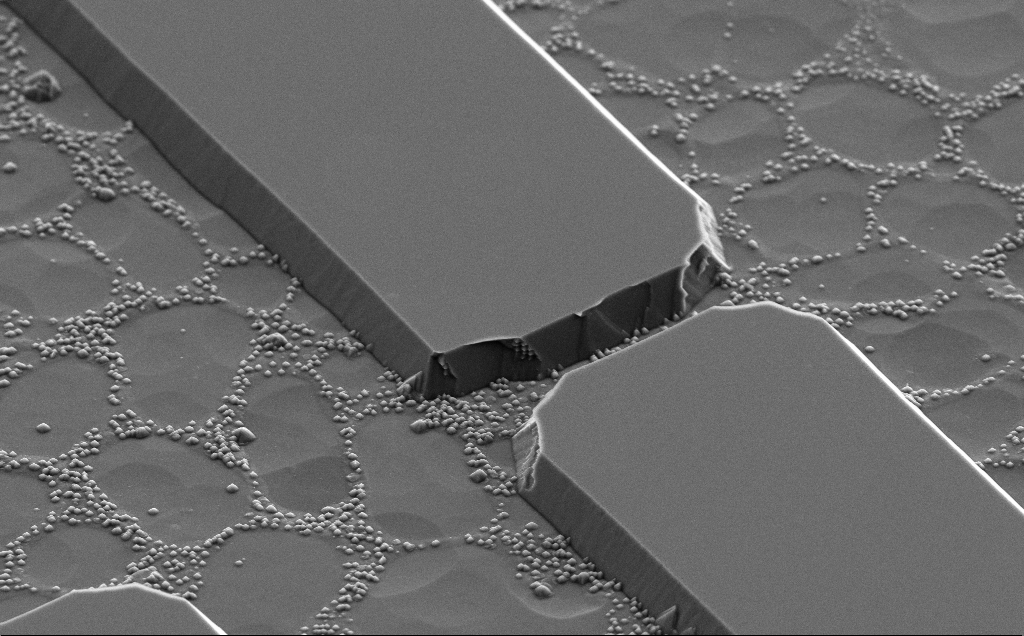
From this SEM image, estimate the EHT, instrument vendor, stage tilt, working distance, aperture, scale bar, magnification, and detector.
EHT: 5 kV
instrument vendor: Zeiss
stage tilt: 50°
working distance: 10 mm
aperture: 30 µm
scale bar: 10000 nm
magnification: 7.01 K X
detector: SE2